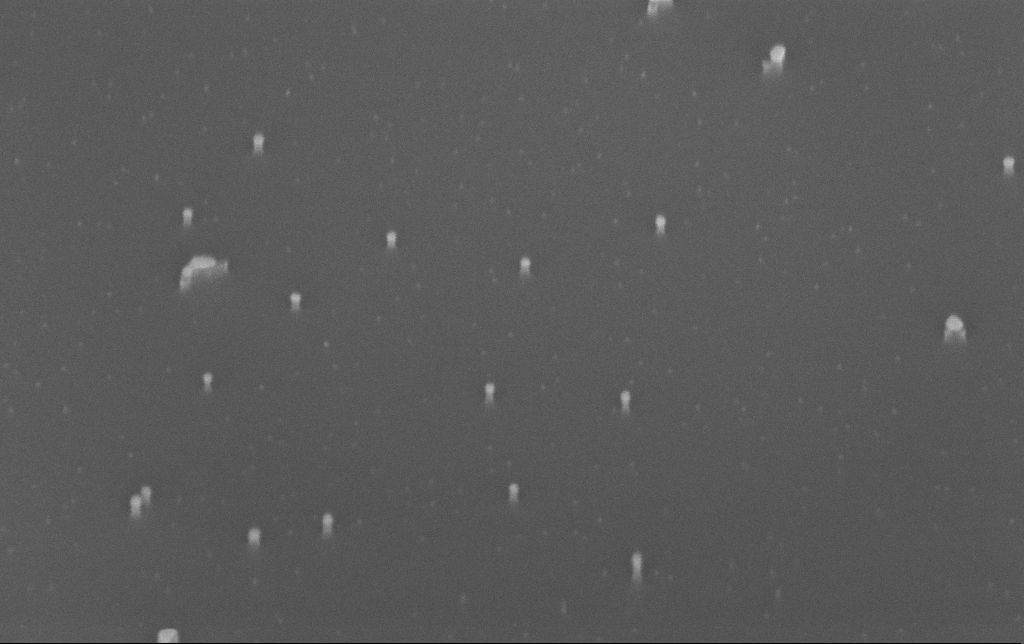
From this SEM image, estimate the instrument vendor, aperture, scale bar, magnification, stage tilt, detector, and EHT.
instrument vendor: Zeiss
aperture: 30 µm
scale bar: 100 nm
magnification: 200 K X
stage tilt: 45°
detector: InLens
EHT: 10 kV